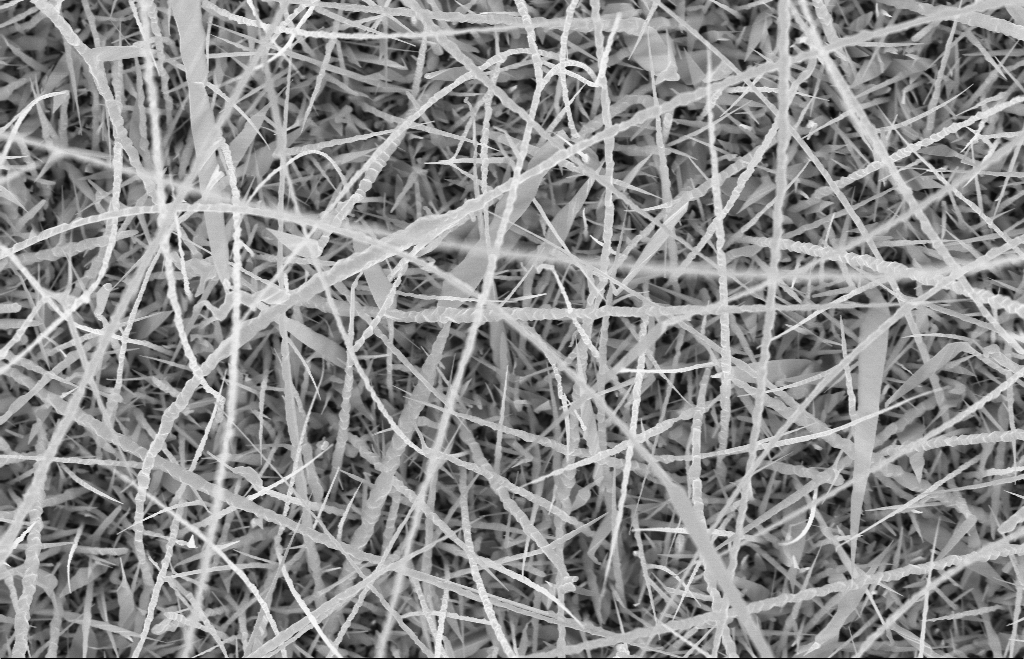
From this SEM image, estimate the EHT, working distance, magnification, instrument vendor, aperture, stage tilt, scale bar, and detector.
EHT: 10 kV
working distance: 9 mm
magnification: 20 K X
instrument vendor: Zeiss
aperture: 30 µm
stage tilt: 0°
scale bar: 1000 nm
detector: InLens